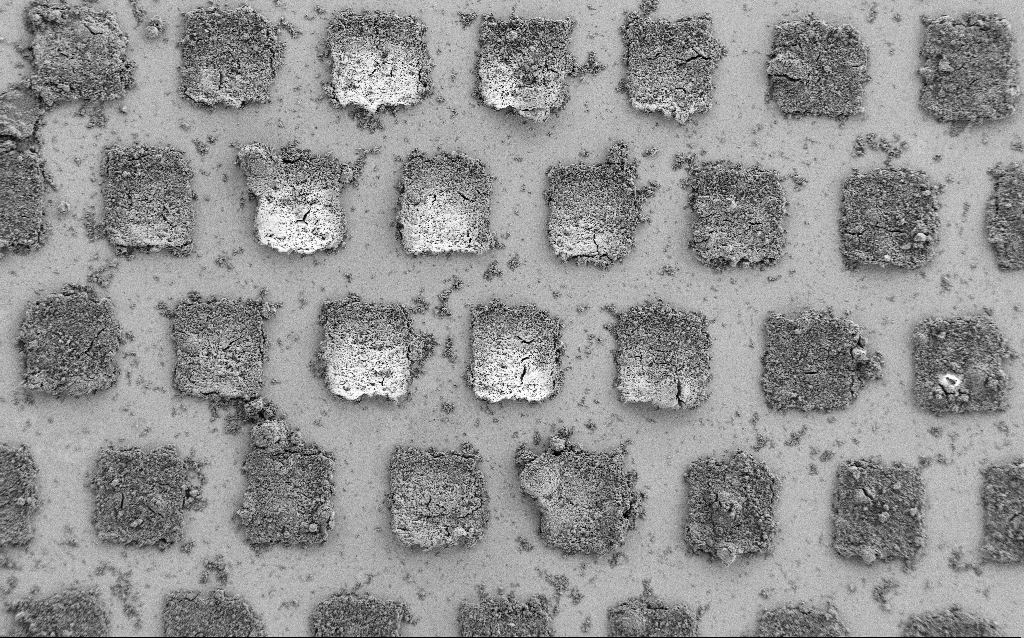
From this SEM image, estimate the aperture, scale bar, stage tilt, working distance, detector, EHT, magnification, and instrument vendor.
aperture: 30 µm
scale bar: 100000 nm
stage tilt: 0°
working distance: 5.6 mm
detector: SE2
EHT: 1.5 kV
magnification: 0.543 K X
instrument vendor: Zeiss